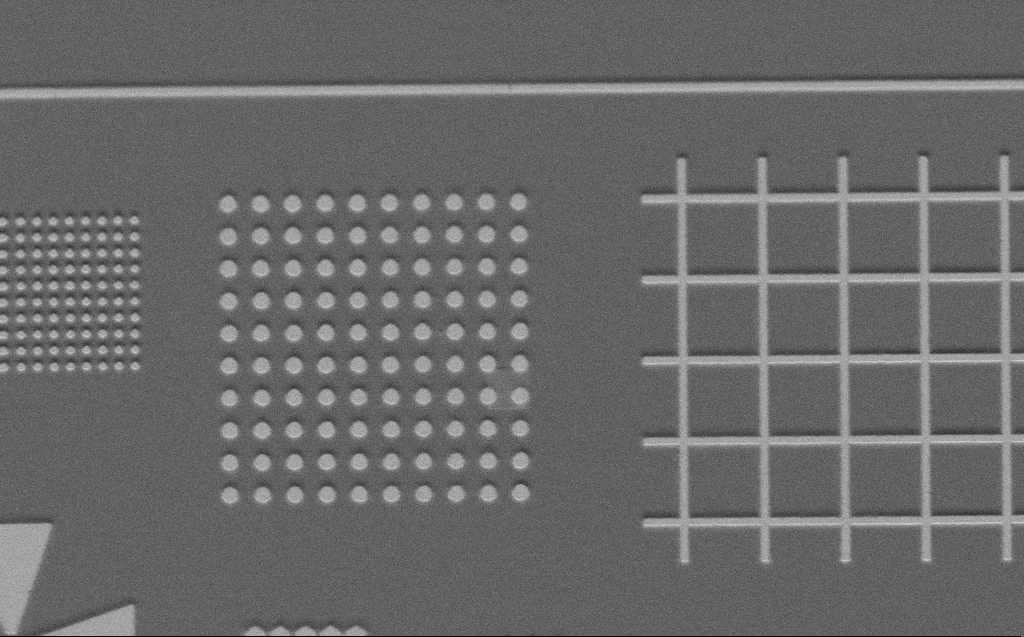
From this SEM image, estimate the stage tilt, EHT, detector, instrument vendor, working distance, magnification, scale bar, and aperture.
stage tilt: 45°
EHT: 3 kV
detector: SE2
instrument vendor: Zeiss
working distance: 6 mm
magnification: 2.97 K X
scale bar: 10000 nm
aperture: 30 µm